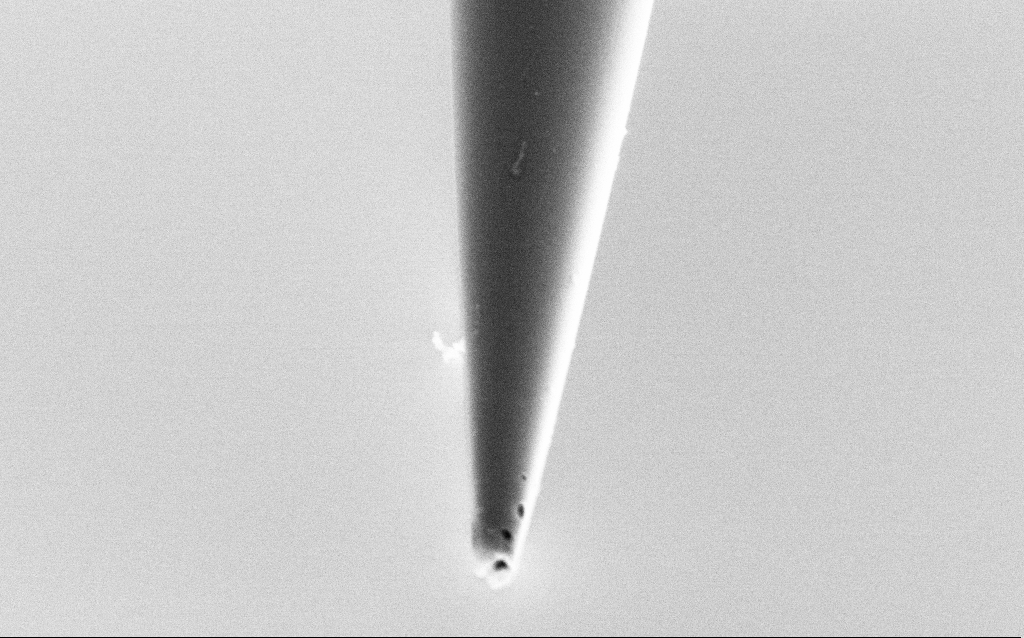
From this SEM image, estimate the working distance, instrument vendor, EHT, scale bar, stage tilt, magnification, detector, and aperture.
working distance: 6 mm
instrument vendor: Zeiss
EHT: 2 kV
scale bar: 1000 nm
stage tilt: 45°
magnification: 50 K X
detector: SE2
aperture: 30 µm